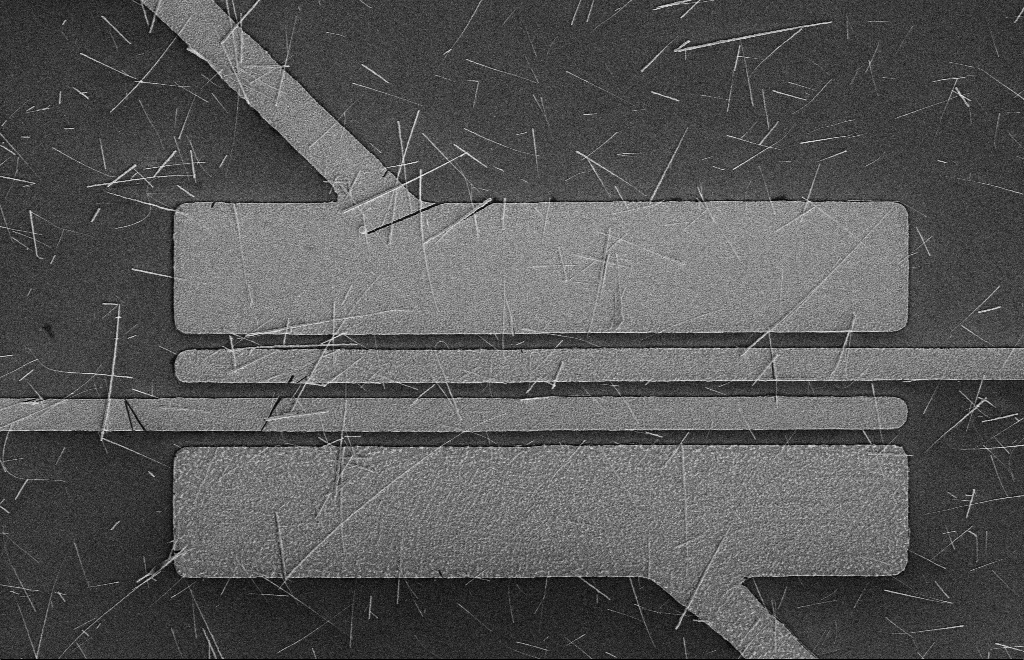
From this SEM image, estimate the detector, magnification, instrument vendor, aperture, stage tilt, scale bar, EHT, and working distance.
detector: SE2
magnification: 4.44 K X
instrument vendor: Zeiss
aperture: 10 µm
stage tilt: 0°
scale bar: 10000 nm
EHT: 5 kV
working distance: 16 mm